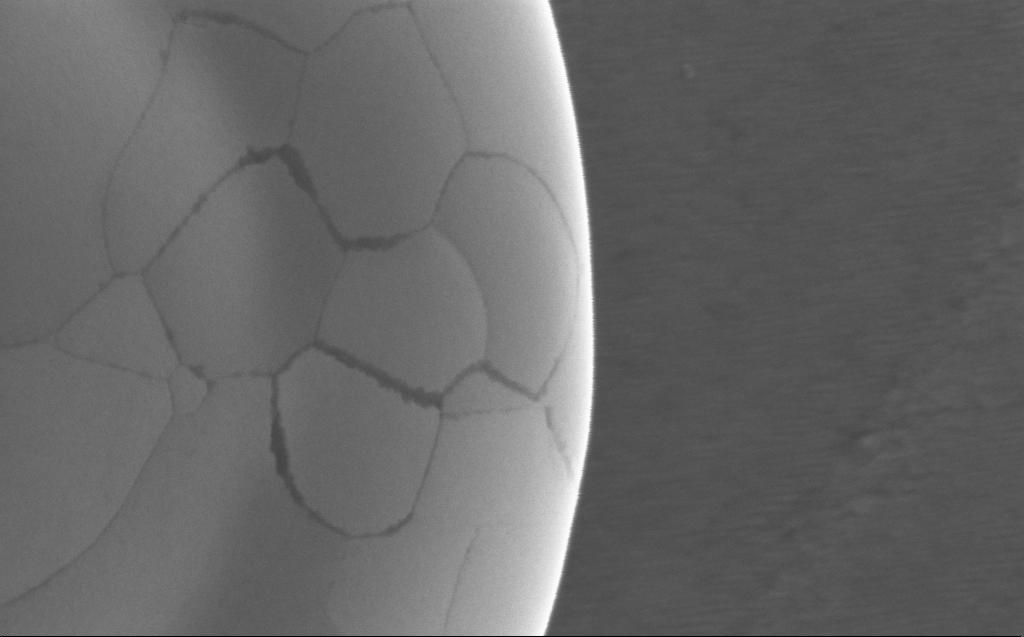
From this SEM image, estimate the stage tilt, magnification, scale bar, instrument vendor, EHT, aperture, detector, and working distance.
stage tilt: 0°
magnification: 185.84 K X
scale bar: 200 nm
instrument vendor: Zeiss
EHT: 5 kV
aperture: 30 µm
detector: InLens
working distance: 2 mm